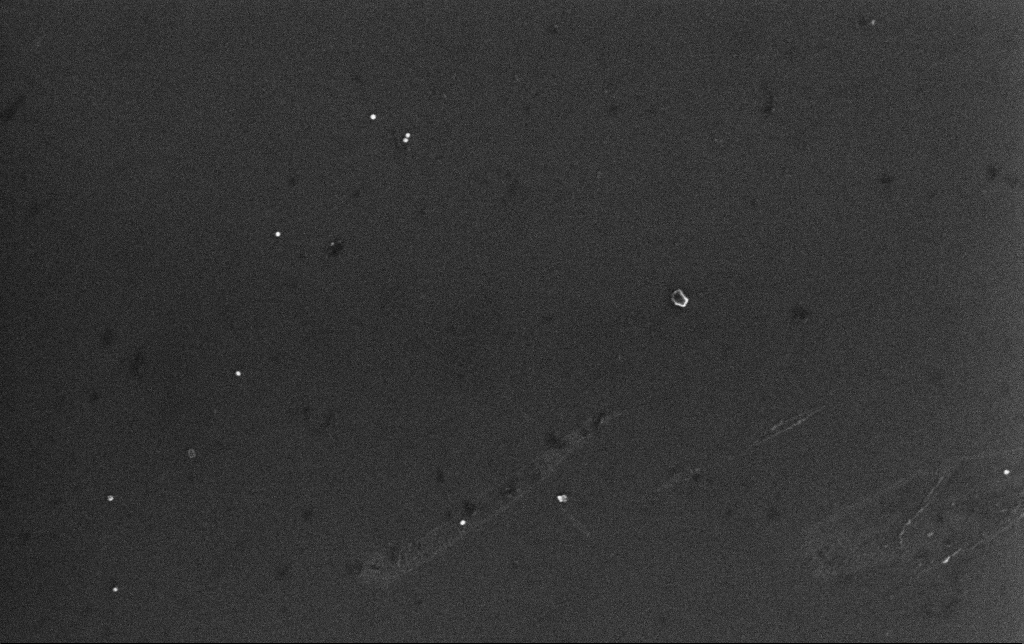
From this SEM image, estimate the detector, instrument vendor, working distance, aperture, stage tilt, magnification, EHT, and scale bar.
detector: InLens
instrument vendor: Zeiss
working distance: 3.4 mm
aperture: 30 µm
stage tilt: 0°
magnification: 100 K X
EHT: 10 kV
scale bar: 200 nm